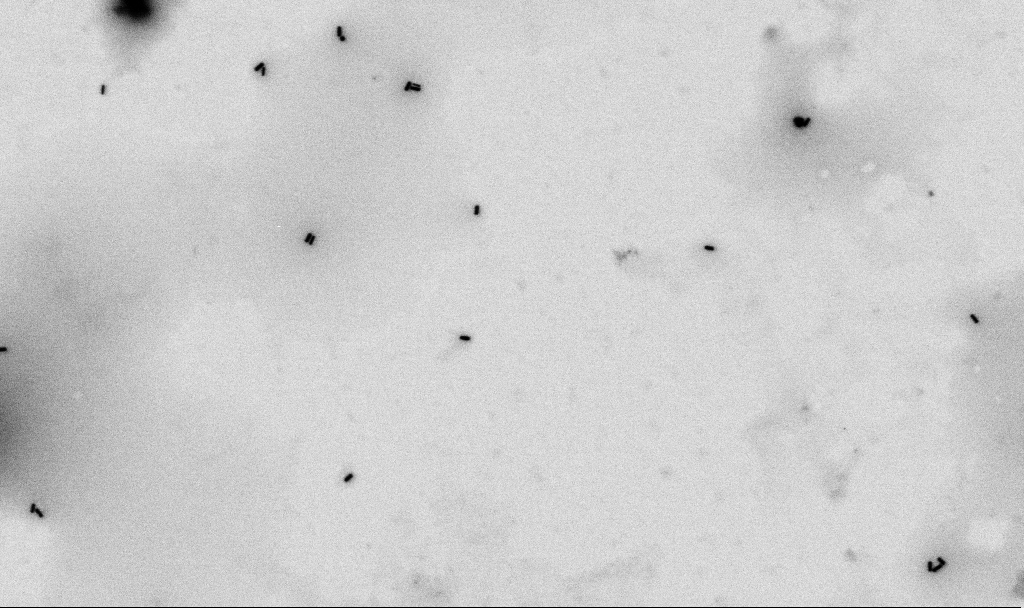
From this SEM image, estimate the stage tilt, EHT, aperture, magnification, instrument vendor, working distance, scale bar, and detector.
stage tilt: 0°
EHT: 2 kV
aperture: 30 µm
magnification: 50 K X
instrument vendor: Zeiss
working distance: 4.5 mm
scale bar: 1000 nm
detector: SE2